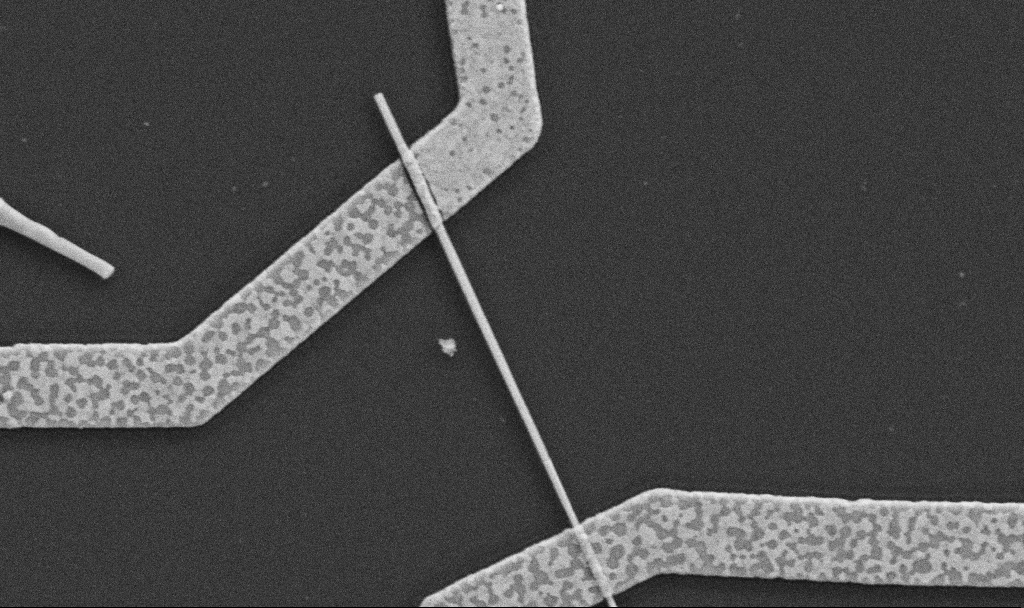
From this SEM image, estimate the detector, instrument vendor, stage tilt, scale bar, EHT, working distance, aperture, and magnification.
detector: SE2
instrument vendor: Zeiss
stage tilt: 0°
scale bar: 1000 nm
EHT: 5 kV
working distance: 8.7 mm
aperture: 30 µm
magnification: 30 K X